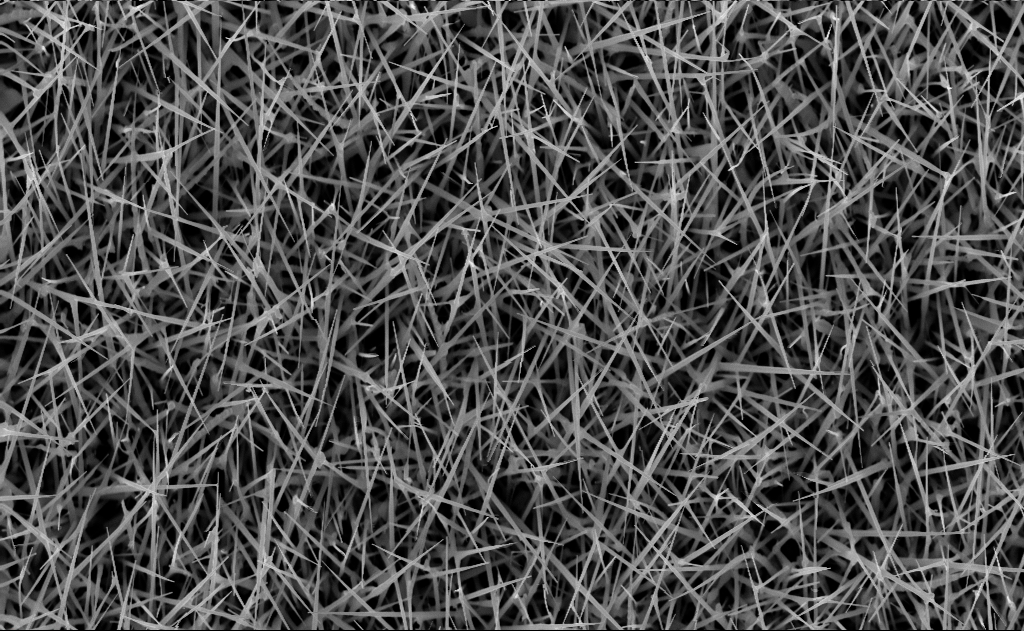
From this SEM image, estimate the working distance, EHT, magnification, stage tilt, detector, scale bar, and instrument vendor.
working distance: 7 mm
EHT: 10 kV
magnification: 20 K X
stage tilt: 0°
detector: InLens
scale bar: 2000 nm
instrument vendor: Zeiss